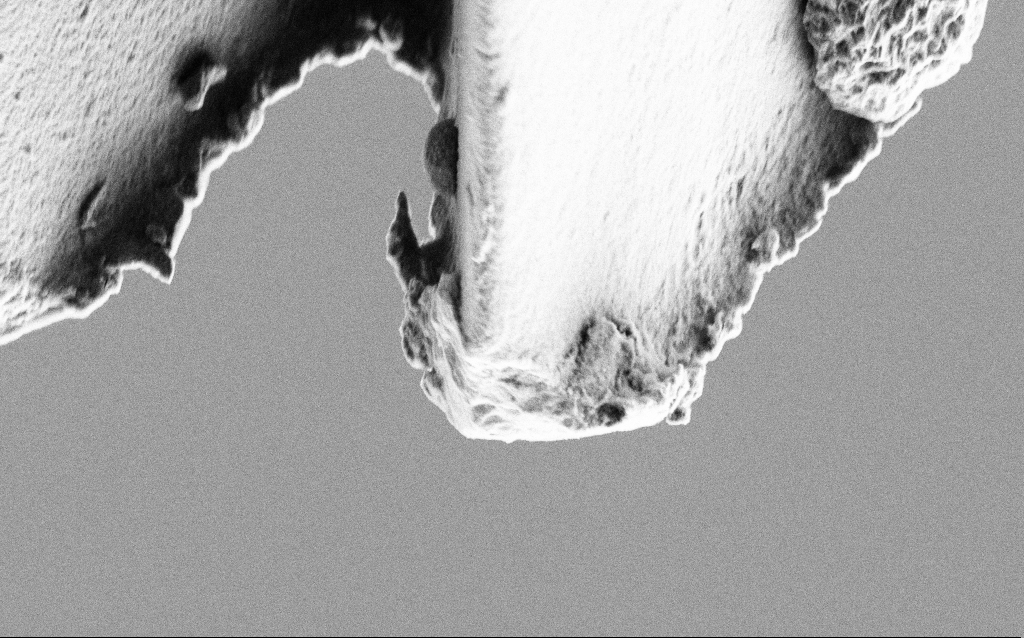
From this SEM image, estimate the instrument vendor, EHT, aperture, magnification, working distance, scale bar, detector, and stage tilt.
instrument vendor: Zeiss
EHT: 3 kV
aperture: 30 µm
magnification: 22.94 K X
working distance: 4 mm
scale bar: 2000 nm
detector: SE2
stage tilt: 45°